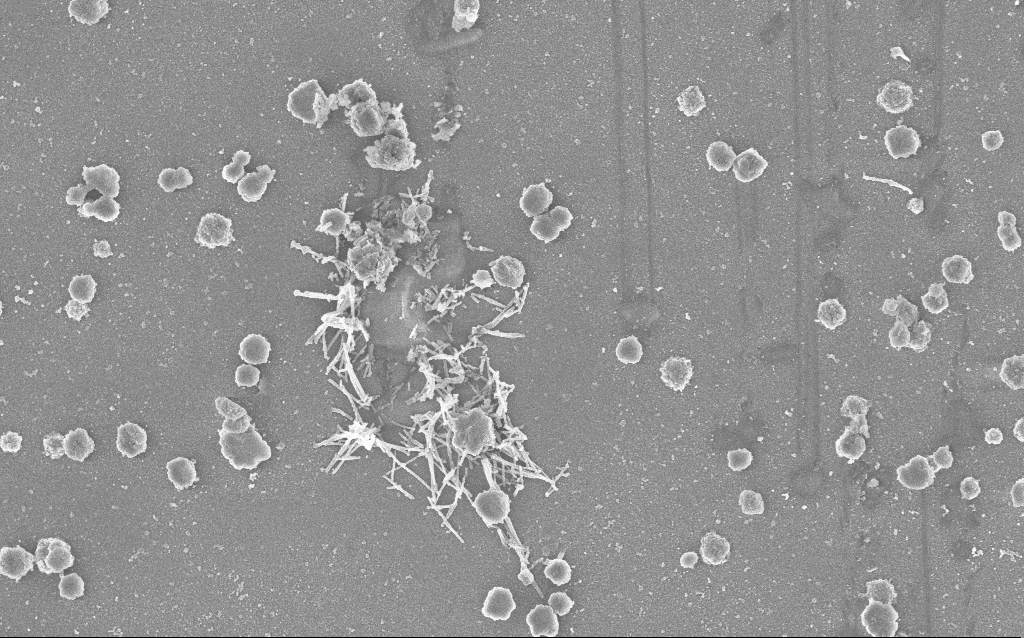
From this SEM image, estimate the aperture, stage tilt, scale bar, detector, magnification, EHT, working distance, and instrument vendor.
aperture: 30 µm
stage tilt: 0°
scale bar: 2000 nm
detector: InLens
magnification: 20 K X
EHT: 3 kV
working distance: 2.5 mm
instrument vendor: Zeiss